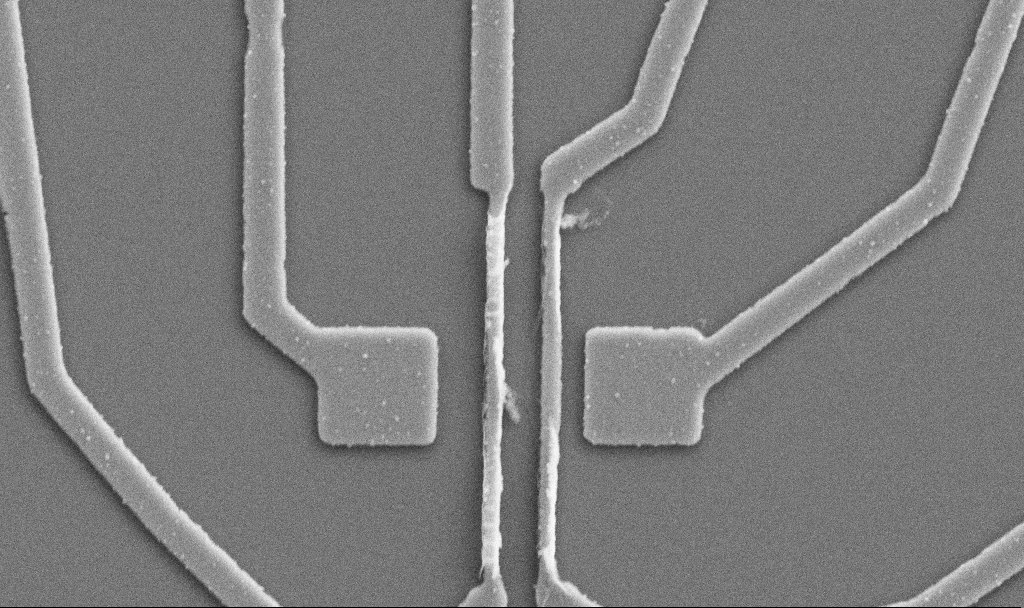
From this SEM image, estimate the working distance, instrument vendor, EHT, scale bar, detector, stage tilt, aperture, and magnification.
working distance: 10.7 mm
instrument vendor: Zeiss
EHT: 5 kV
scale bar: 1000 nm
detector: SE2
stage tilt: -0°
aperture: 30 µm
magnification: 20 K X